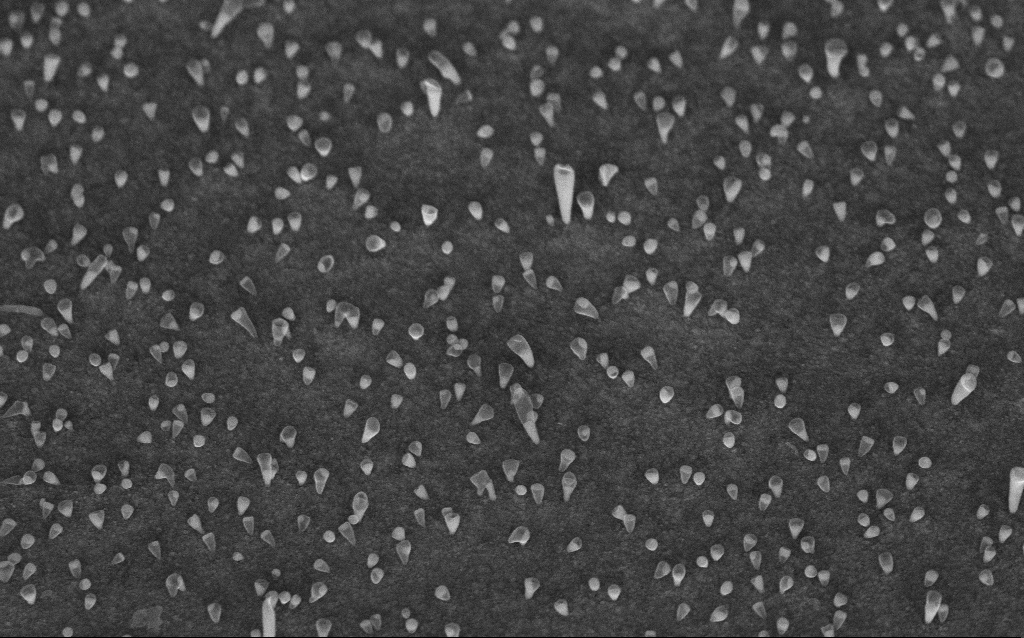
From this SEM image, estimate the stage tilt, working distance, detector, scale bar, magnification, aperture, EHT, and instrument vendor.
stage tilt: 45°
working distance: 6.6 mm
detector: InLens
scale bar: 1000 nm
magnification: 50 K X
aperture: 30 µm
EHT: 5 kV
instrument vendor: Zeiss